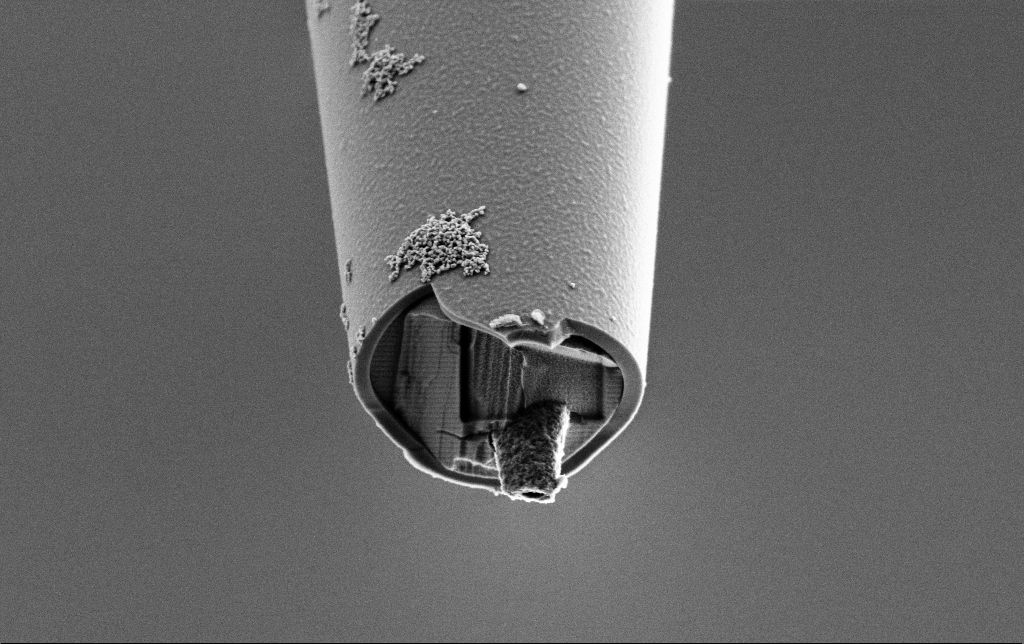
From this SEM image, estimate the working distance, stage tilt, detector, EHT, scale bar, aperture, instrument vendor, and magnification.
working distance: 7.3 mm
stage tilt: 45°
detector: SE2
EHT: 2 kV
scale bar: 1000 nm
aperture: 30 µm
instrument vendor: Zeiss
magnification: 25 K X